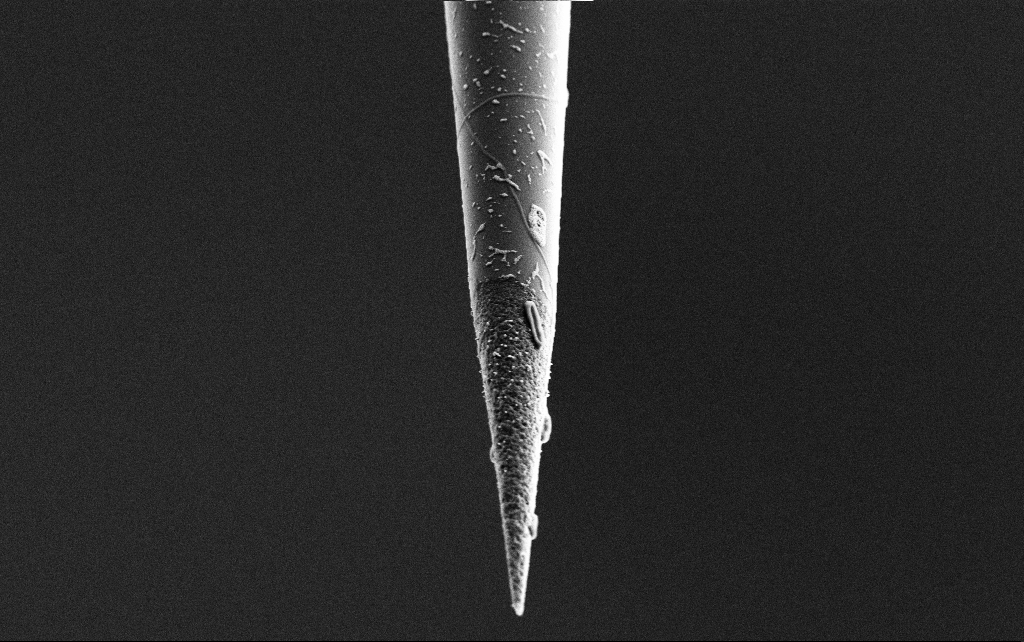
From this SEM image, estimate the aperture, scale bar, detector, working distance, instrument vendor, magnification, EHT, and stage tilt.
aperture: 30 µm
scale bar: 2000 nm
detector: SE2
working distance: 7.7 mm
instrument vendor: Zeiss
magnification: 10 K X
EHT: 3 kV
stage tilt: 45°